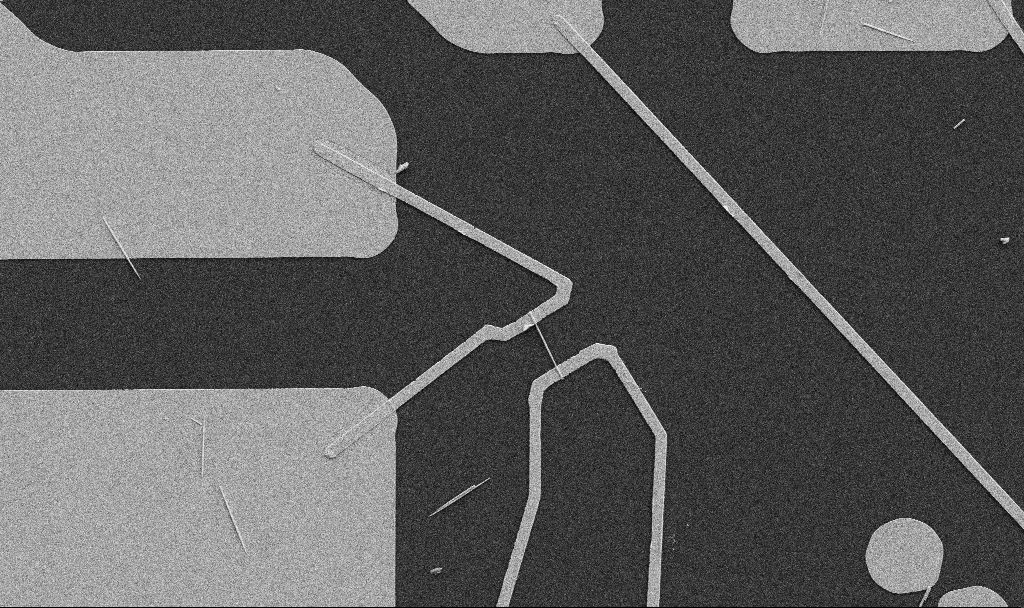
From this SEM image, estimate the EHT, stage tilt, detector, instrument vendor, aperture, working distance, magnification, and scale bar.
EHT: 5 kV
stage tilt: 0°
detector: SE2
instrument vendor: Zeiss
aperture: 30 µm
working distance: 10.7 mm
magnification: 5 K X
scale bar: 10000 nm